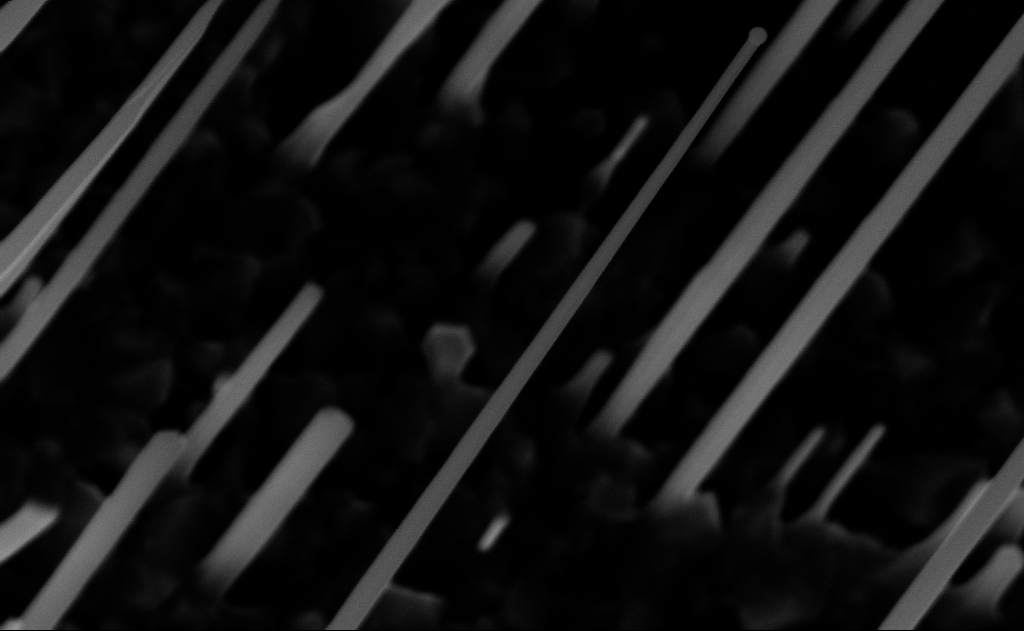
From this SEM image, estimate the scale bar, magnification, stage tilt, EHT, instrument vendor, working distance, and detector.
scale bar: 200 nm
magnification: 80 K X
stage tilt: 0°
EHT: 10 kV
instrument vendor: Zeiss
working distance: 10 mm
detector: InLens